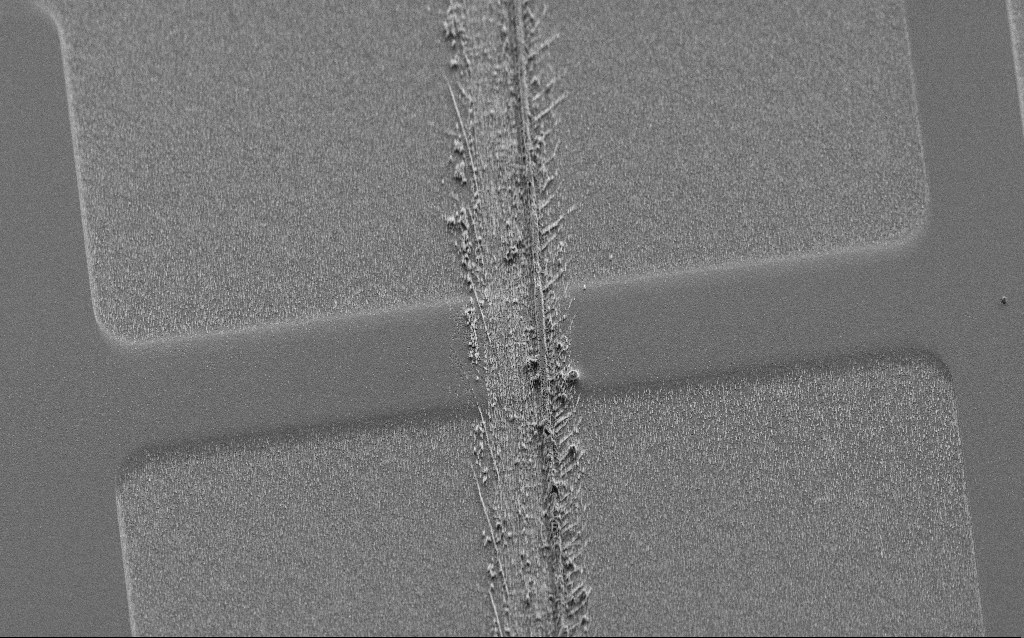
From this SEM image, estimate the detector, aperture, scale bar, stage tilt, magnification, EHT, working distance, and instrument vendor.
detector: SE2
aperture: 30 µm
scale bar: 20000 nm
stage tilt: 45°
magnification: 0.773 K X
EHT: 5 kV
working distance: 8 mm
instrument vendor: Zeiss